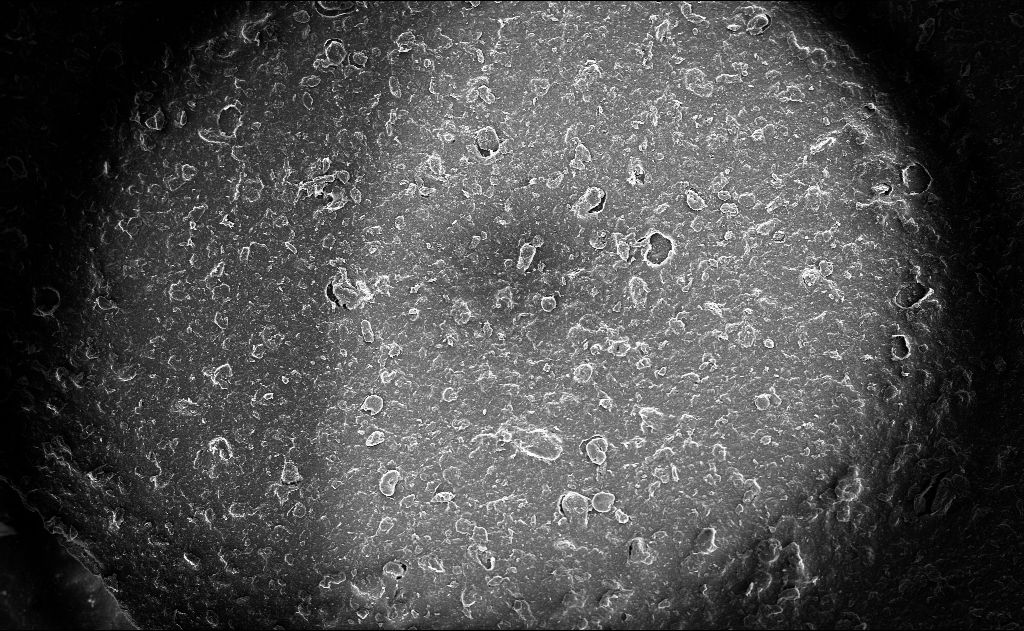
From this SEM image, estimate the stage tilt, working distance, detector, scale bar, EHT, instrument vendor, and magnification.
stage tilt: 0°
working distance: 3 mm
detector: InLens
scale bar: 200000 nm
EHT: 10 kV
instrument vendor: Zeiss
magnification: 0.119 K X